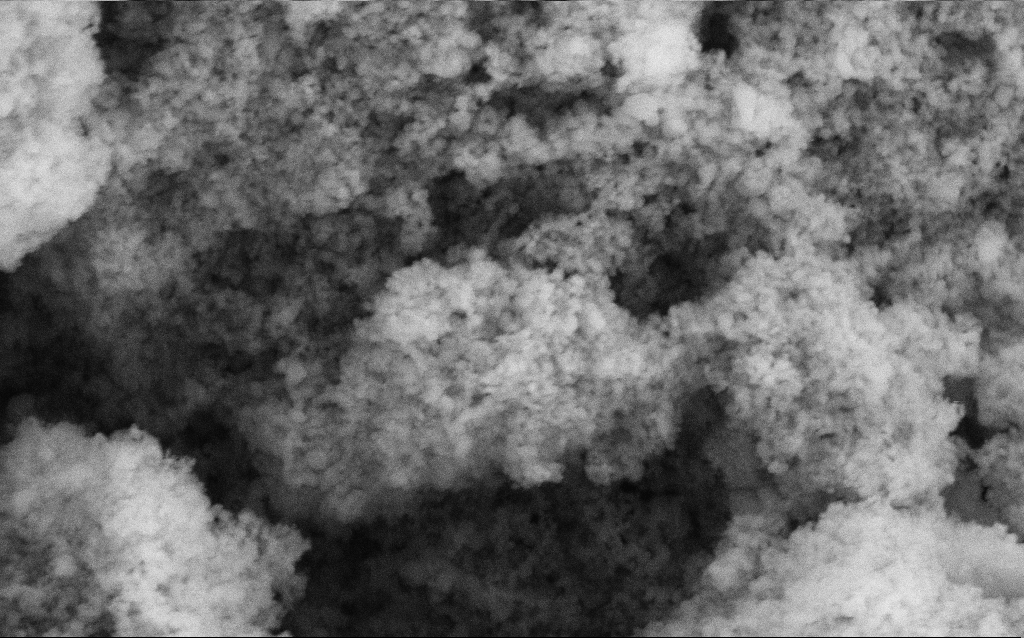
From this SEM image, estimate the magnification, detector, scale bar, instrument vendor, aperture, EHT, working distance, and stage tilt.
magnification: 114.25 K X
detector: SE2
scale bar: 200 nm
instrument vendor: Zeiss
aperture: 30 µm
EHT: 5 kV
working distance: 4.6 mm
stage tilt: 0°